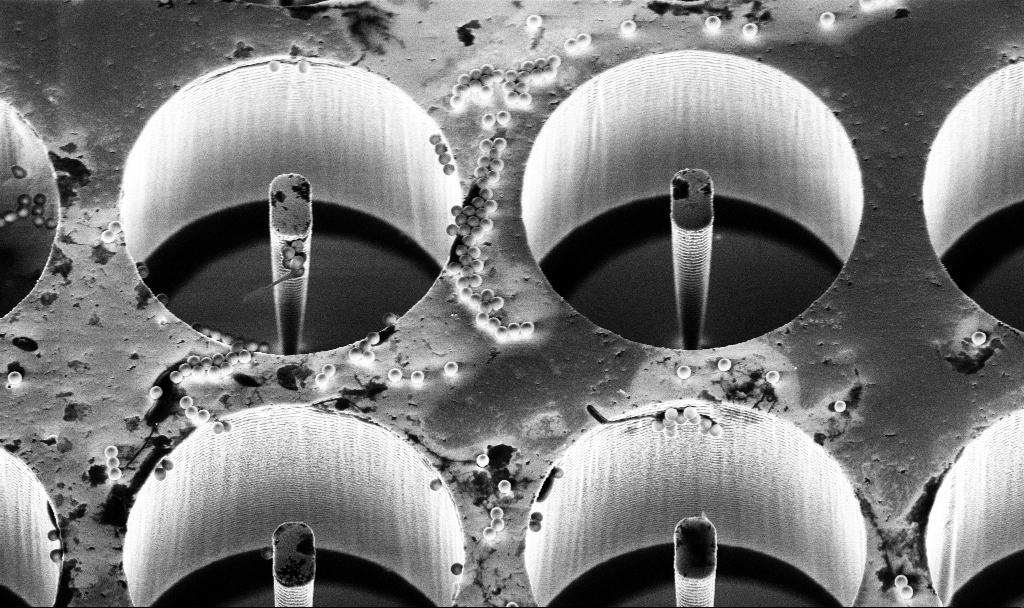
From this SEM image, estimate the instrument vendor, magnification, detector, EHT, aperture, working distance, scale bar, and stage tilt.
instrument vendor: Zeiss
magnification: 6.42 K X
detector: InLens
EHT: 5 kV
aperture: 30 µm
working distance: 7.1 mm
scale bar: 10000 nm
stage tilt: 30°